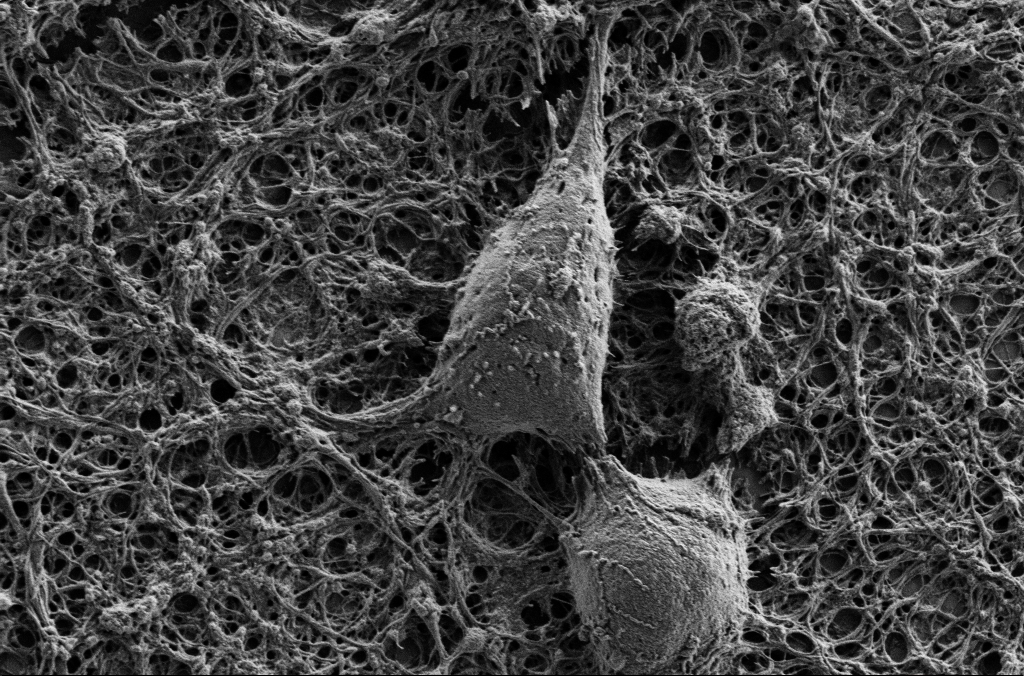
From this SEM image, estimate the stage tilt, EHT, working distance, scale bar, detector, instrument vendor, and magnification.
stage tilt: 0°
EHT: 1 kV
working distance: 4.1 mm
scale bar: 2000 nm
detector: SE2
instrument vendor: Zeiss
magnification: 10 K X